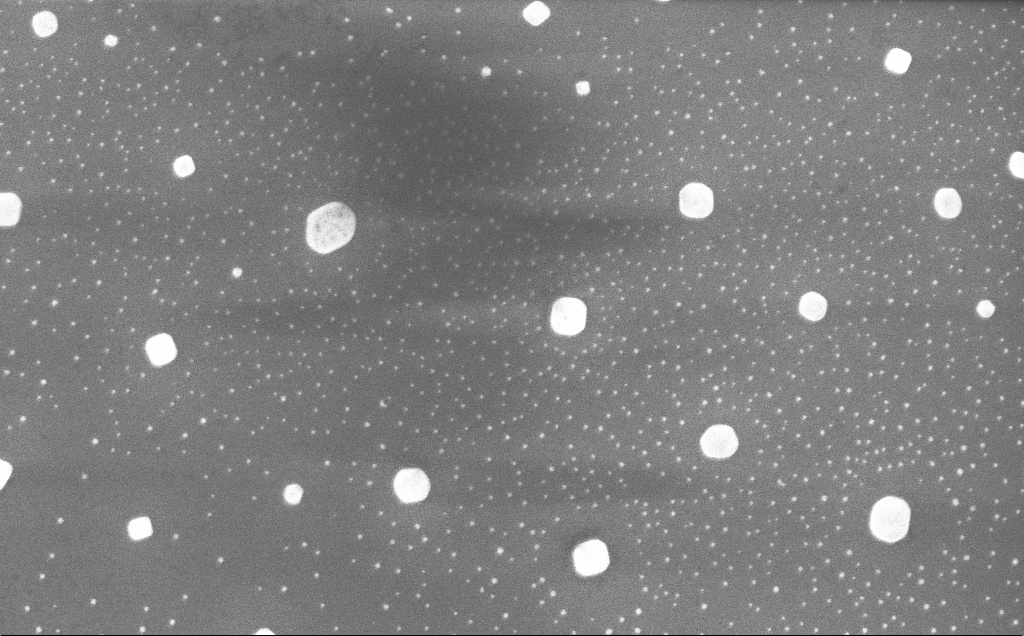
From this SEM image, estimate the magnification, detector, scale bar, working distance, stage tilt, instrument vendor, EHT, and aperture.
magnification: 40 K X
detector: InLens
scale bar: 1000 nm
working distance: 4 mm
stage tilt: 0°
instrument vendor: Zeiss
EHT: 10 kV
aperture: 30 µm